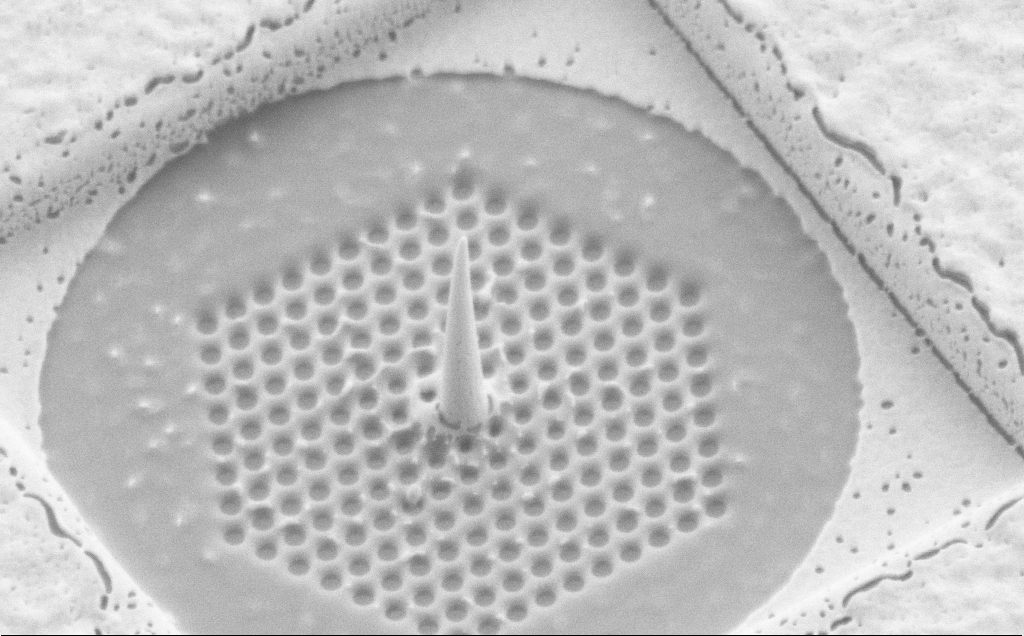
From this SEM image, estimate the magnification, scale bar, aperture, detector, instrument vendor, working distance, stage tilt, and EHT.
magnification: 49.69 K X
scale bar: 1000 nm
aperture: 30 µm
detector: SE2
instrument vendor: Zeiss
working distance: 6 mm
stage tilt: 34.3°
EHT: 3 kV